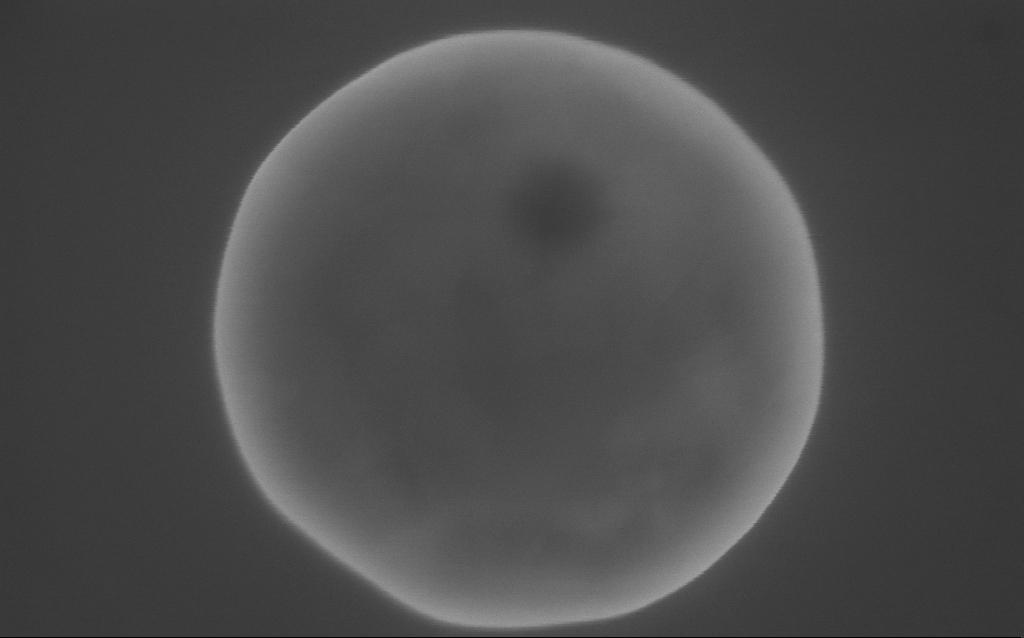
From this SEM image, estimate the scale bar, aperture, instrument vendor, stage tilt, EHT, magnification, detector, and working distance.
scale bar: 200 nm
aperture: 30 µm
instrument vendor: Zeiss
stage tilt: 0°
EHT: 10 kV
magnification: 175.69 K X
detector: InLens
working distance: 2 mm